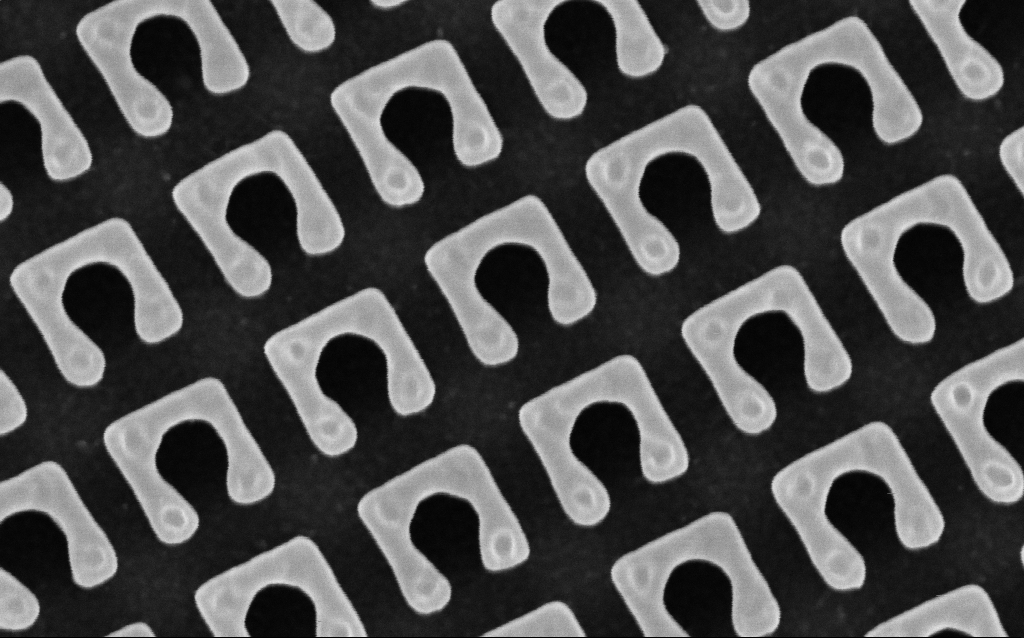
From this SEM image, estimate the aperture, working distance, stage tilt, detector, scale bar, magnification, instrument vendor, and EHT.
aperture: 30 µm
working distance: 4.6 mm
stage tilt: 0°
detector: InLens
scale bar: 200 nm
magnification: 148.69 K X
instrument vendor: Zeiss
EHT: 3 kV